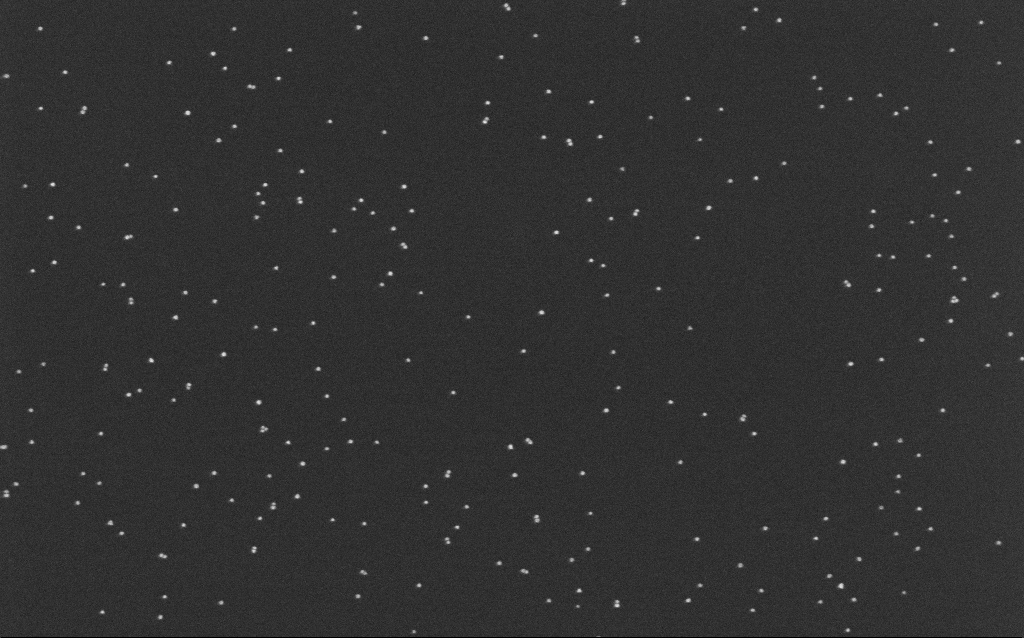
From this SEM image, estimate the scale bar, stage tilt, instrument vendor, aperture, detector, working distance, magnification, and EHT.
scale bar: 200 nm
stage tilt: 0°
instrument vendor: Zeiss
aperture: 30 µm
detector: InLens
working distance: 6.6 mm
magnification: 100 K X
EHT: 10 kV